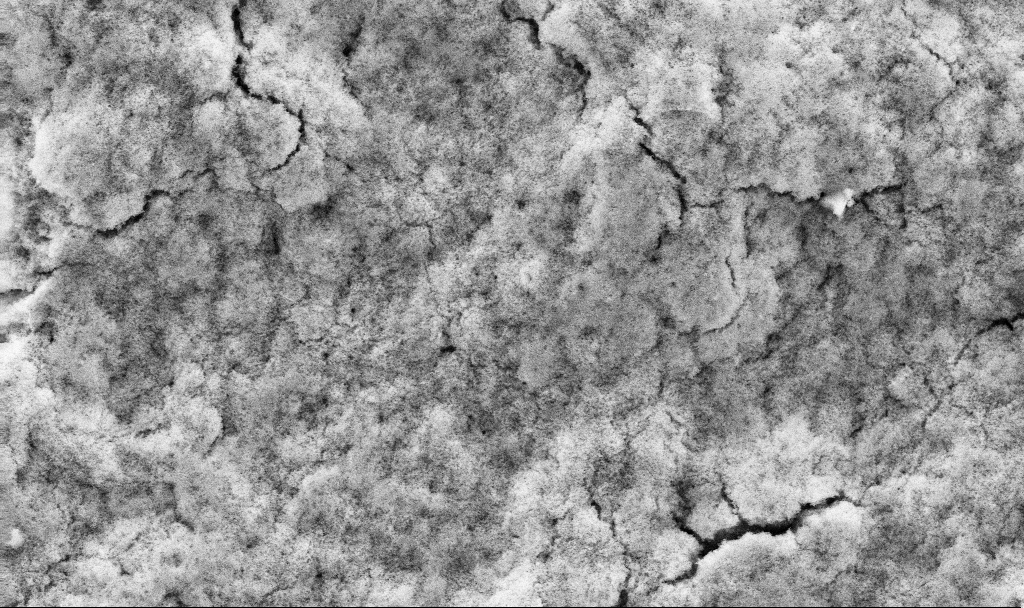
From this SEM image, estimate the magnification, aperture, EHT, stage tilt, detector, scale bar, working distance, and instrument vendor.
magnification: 5.53 K X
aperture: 30 µm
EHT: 10 kV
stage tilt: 0°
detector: SE2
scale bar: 10000 nm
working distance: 2.7 mm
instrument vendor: Zeiss